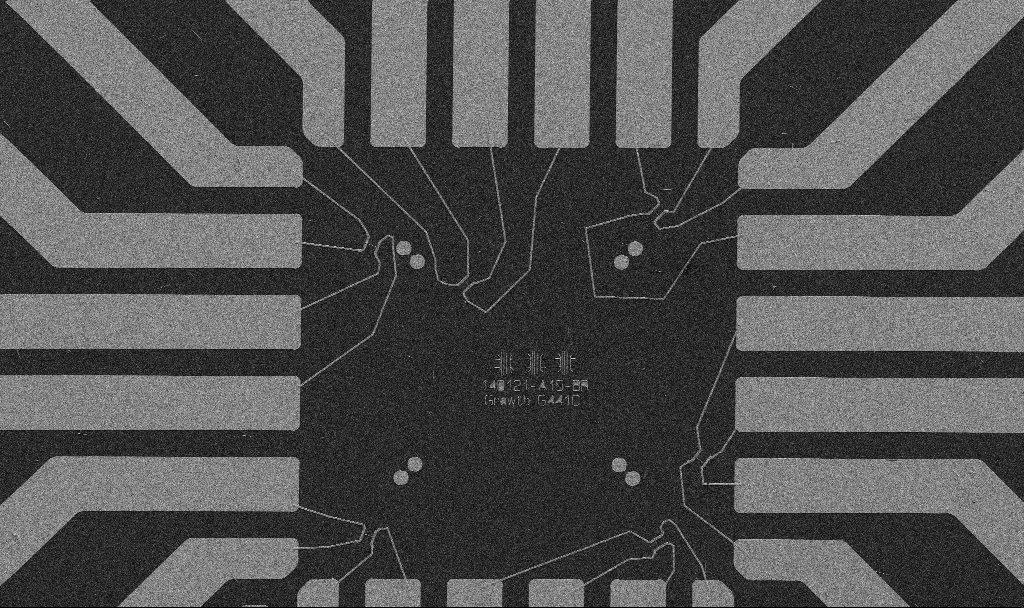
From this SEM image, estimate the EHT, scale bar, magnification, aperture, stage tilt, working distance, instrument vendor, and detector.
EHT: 5 kV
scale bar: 20000 nm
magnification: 1 K X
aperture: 30 µm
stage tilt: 0°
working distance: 10.7 mm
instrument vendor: Zeiss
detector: SE2